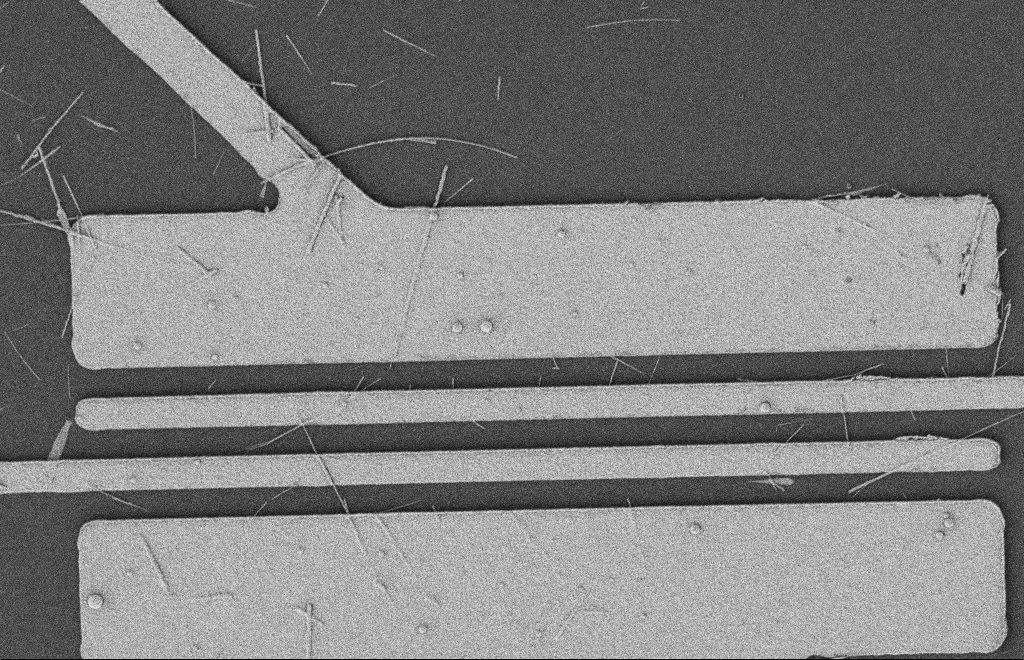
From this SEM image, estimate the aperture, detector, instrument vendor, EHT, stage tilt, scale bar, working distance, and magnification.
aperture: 20 µm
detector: SE2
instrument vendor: Zeiss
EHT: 2 kV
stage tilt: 0°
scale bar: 2000 nm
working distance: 12 mm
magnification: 5.56 K X